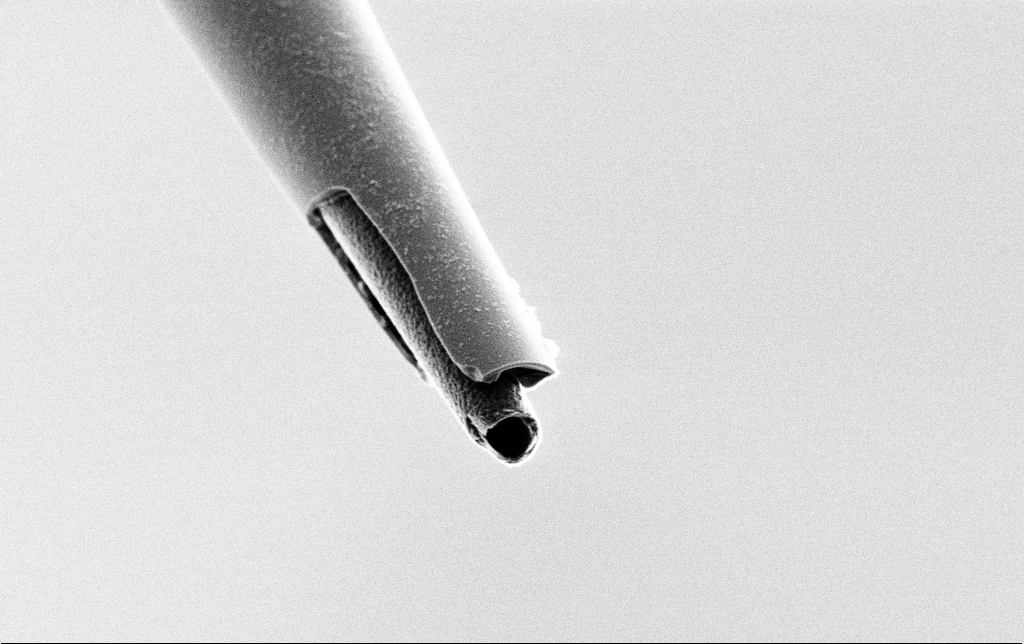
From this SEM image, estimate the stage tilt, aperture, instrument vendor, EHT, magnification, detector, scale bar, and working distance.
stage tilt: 45°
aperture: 30 µm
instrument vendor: Zeiss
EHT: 3 kV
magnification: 10 K X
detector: SE2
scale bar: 2000 nm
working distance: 7.4 mm